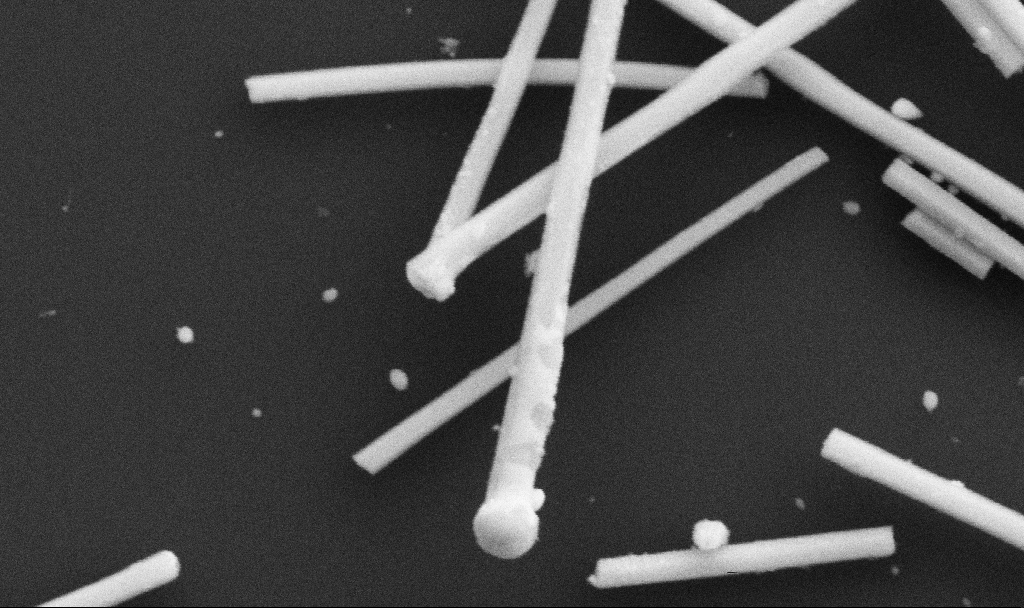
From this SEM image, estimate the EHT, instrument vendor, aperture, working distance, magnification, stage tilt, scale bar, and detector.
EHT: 5 kV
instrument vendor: Zeiss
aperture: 30 µm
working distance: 10.7 mm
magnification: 106.66 K X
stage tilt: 0°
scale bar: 200 nm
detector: SE2